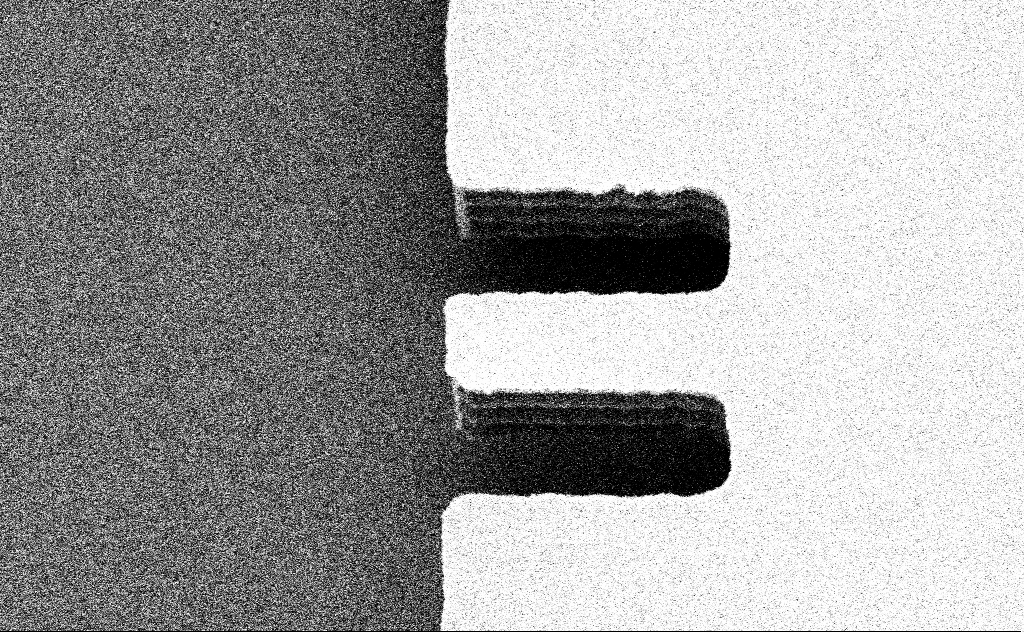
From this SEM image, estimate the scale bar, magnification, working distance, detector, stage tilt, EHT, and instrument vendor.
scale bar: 10000 nm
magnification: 7.12 K X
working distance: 10 mm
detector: SE2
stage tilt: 43°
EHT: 5 kV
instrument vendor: Zeiss